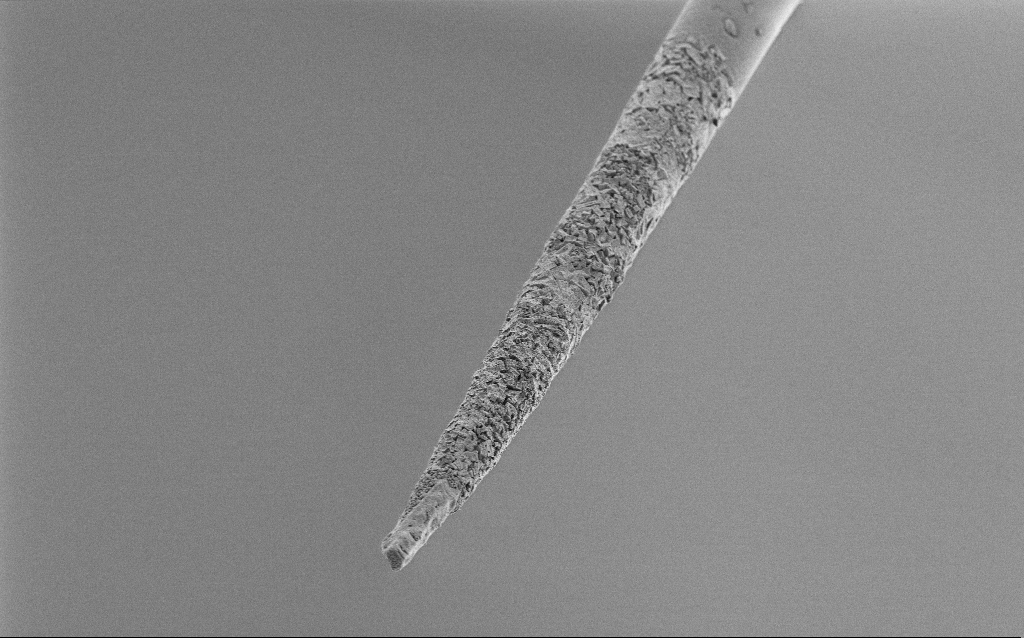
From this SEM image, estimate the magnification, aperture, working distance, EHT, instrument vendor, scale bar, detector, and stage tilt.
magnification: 1.5 K X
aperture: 30 µm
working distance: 6.8 mm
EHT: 1 kV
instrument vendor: Zeiss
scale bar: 10000 nm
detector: SE2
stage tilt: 45°